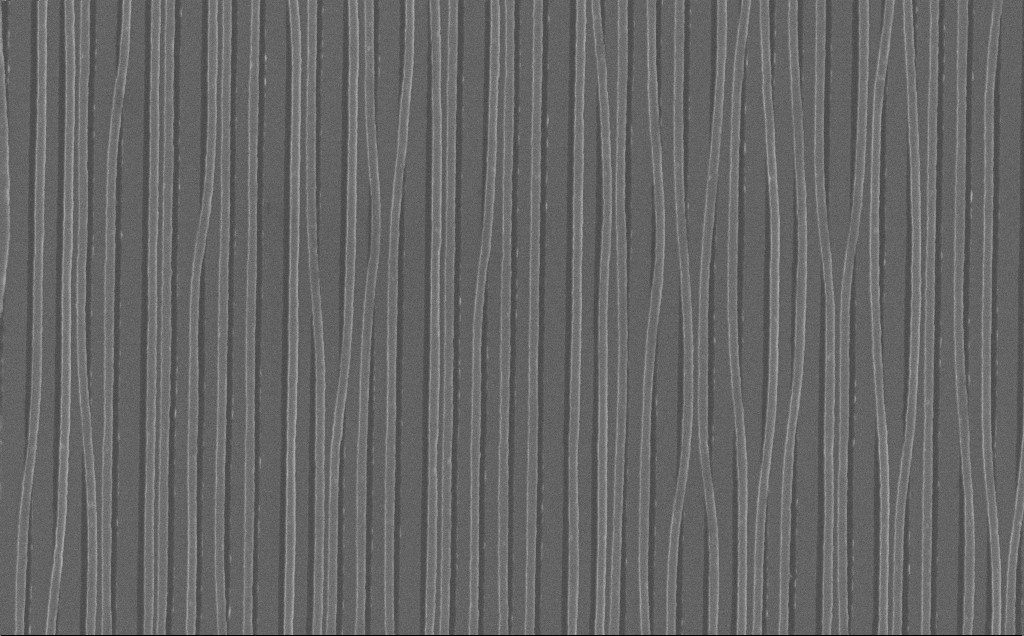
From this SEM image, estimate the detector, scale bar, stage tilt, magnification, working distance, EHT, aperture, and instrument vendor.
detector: InLens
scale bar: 2000 nm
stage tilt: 0°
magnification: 26.01 K X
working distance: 7 mm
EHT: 10 kV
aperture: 30 µm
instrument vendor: Zeiss